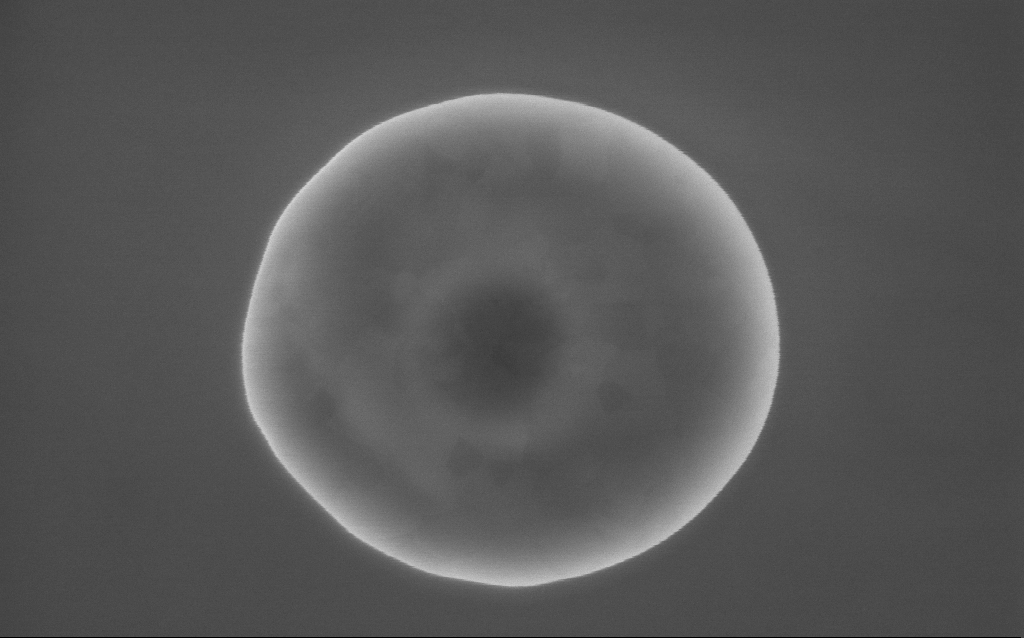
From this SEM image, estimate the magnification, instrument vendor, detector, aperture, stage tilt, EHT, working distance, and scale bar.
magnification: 157 K X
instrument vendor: Zeiss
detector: InLens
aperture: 30 µm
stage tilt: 0°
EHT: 10 kV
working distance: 2 mm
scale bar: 100 nm